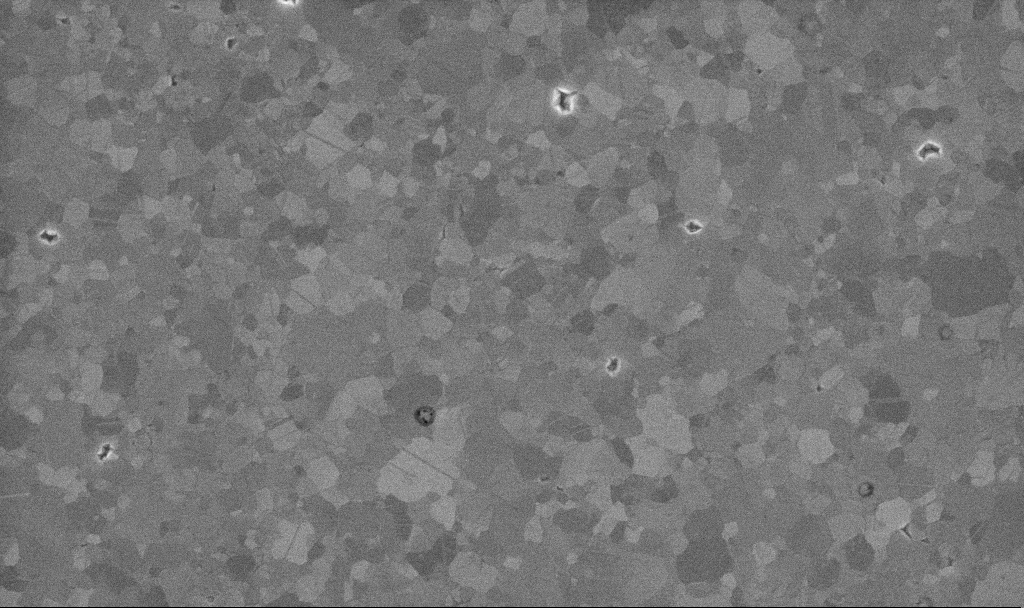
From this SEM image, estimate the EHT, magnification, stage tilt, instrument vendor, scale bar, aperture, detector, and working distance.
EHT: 10 kV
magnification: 50.87 K X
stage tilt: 0°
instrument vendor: Zeiss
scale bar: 1000 nm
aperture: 30 µm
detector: InLens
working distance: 3.3 mm